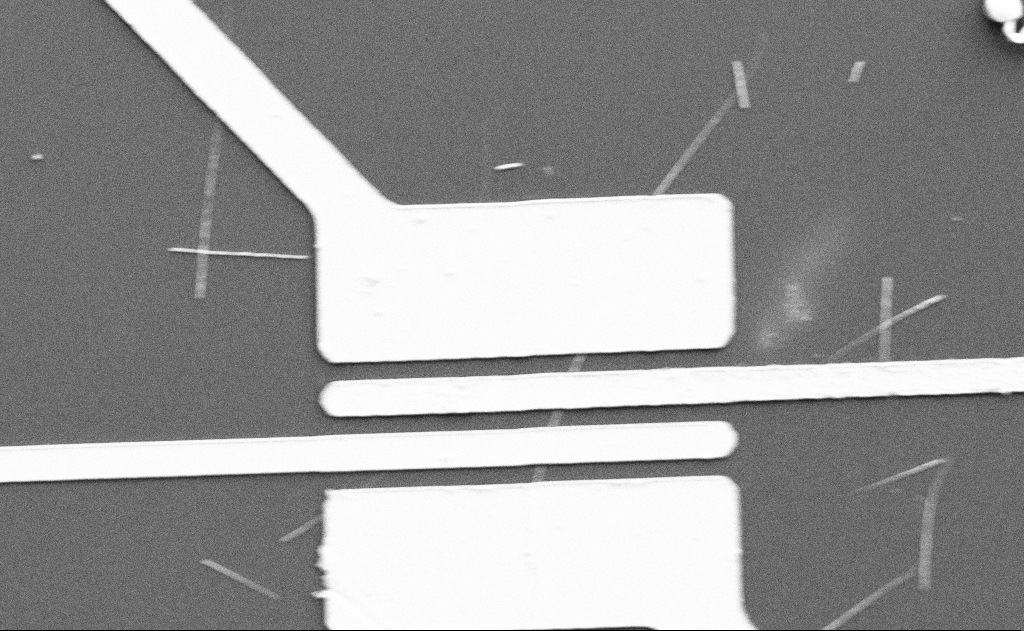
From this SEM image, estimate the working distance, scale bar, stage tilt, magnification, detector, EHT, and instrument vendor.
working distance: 15 mm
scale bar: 10000 nm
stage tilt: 0°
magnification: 5 K X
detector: SE2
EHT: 5 kV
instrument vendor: Zeiss